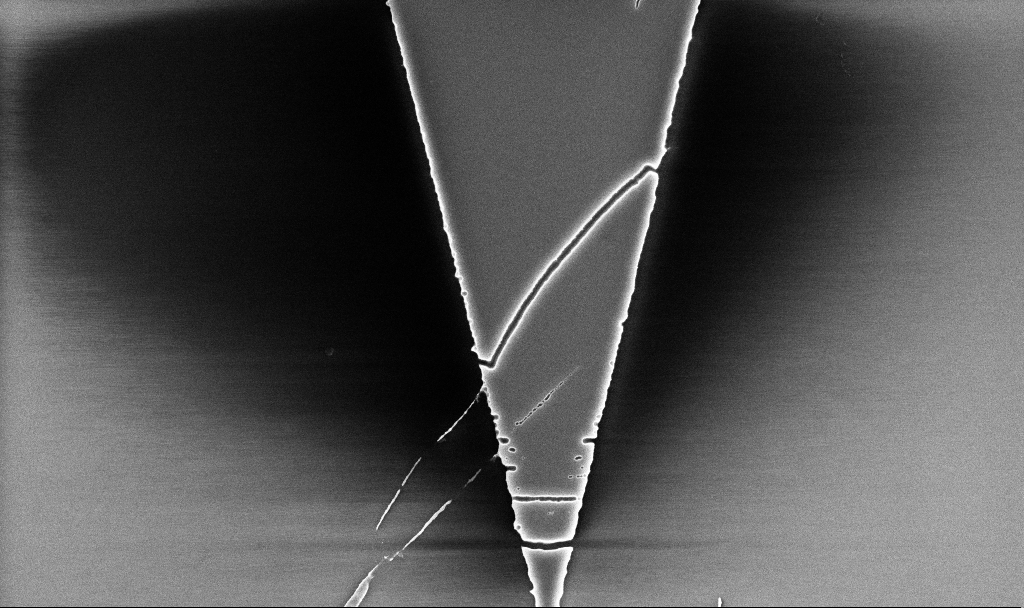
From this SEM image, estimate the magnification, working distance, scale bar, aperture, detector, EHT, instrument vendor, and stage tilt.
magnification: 17.2 K X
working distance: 5.2 mm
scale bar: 2000 nm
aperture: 30 µm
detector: InLens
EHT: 5 kV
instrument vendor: Zeiss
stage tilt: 0°